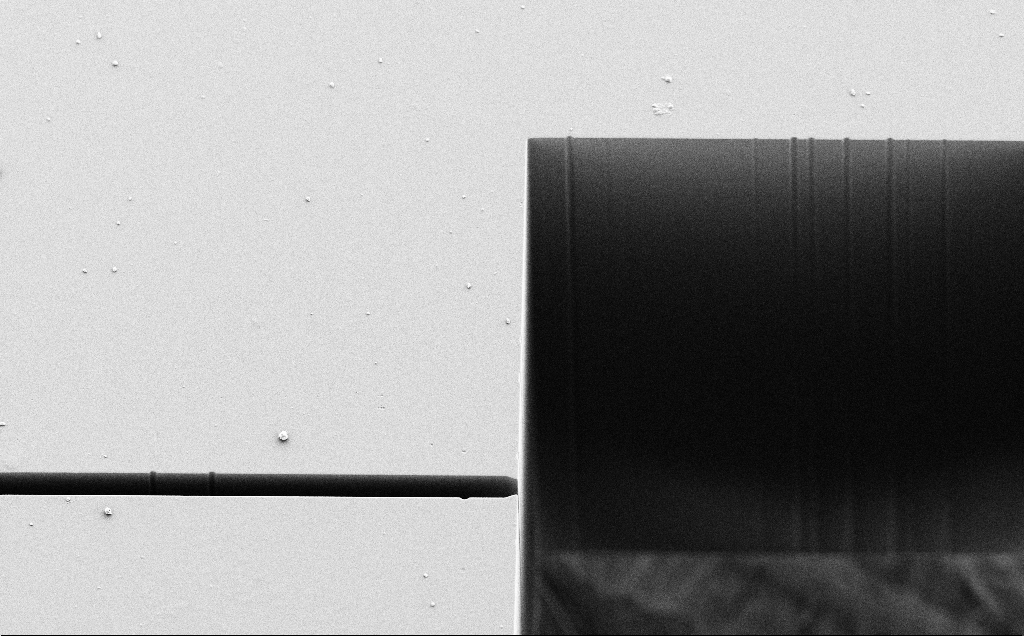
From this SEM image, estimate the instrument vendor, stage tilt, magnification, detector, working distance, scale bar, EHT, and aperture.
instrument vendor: Zeiss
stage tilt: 25°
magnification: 0.658 K X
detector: SE2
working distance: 7 mm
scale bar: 100000 nm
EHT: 5 kV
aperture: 30 µm